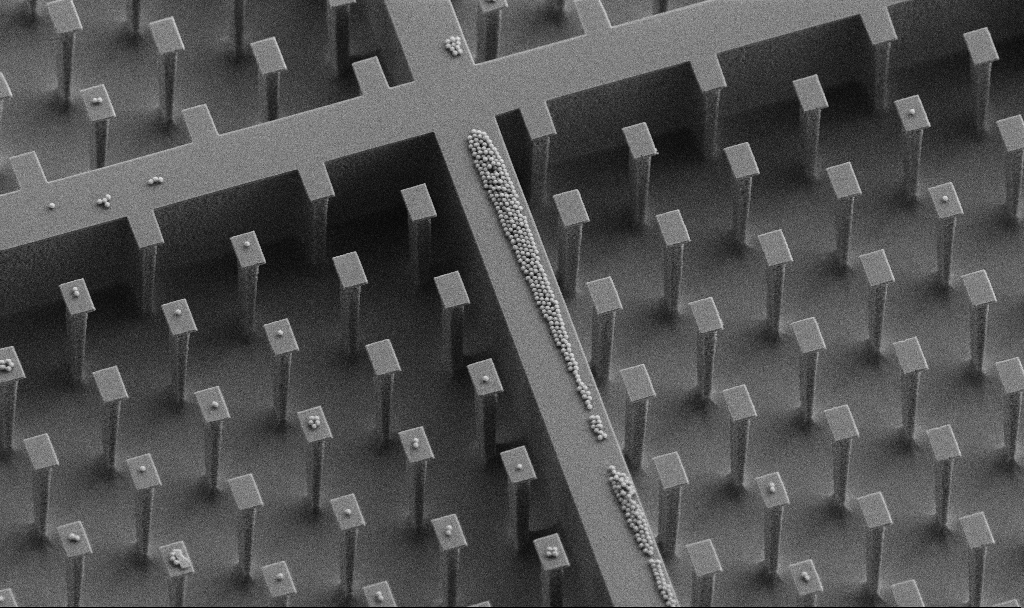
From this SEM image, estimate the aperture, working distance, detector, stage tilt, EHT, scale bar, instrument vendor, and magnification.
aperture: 30 µm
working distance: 4.5 mm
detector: SE2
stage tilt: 30°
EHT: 5 kV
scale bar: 10000 nm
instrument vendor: Zeiss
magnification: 3.3 K X